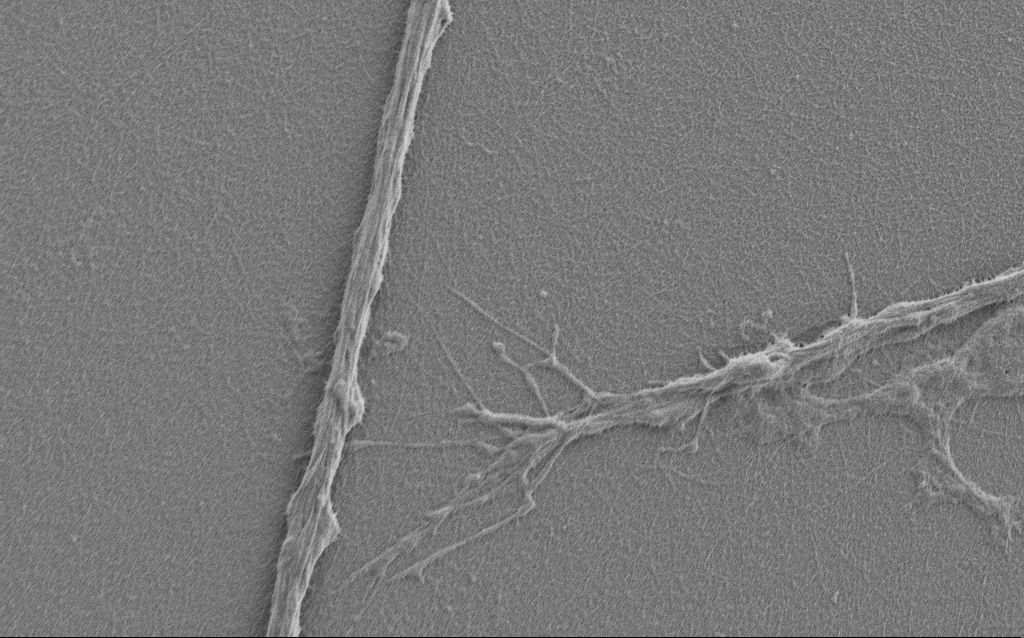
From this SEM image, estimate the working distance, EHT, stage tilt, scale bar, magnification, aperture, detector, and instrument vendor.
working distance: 6 mm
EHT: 1 kV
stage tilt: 0°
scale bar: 2000 nm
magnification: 7.5 K X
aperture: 30 µm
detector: SE2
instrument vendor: Zeiss